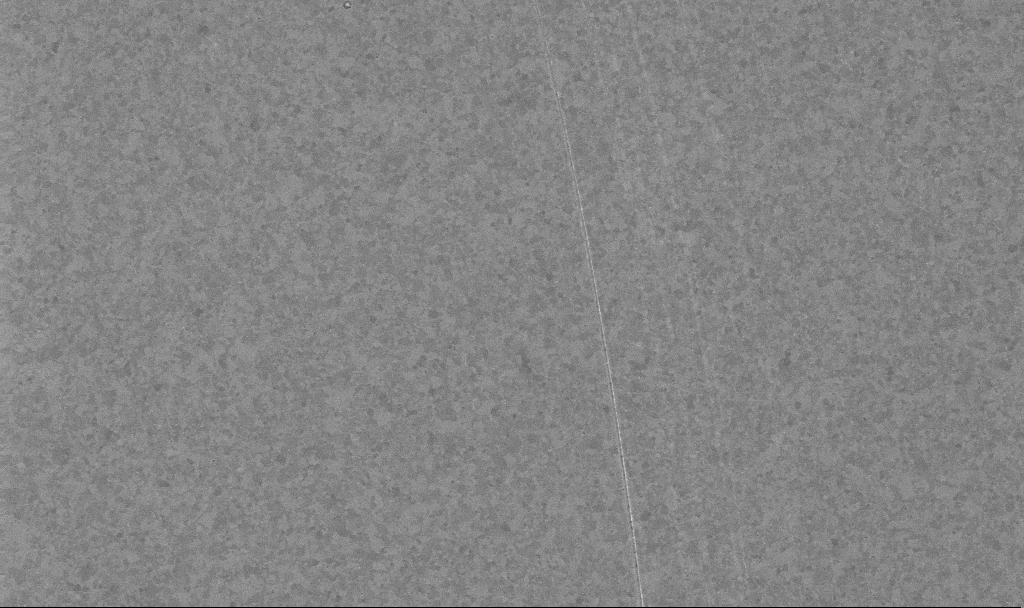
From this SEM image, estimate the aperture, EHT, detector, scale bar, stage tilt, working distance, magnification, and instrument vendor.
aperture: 30 µm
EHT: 10 kV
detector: InLens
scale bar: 2000 nm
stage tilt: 0°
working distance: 3.4 mm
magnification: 10 K X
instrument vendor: Zeiss